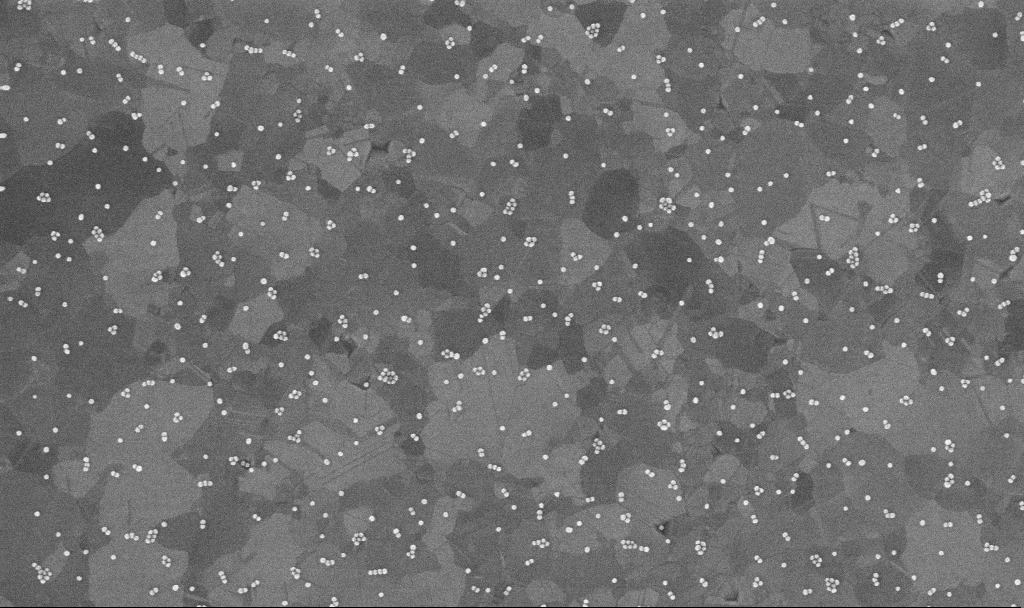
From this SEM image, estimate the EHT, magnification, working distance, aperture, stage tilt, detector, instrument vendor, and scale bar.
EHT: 10 kV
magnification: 100 K X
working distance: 3.4 mm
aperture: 30 µm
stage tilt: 0°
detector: InLens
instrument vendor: Zeiss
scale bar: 200 nm